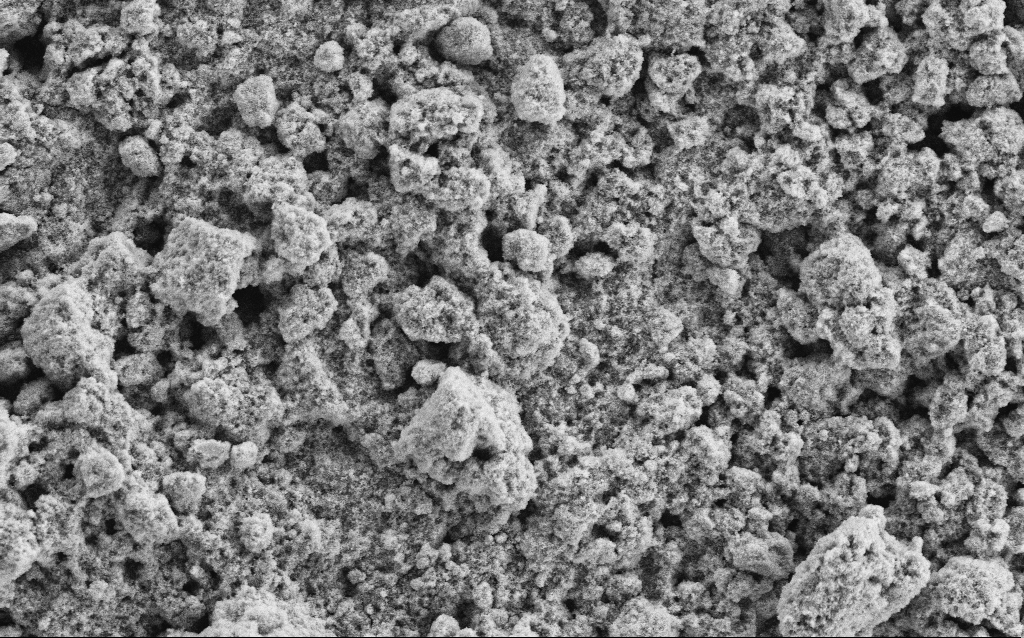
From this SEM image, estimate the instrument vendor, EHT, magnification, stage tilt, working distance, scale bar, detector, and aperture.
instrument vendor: Zeiss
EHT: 5 kV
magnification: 6.45 K X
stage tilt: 0°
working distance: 4.6 mm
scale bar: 10000 nm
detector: SE2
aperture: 30 µm